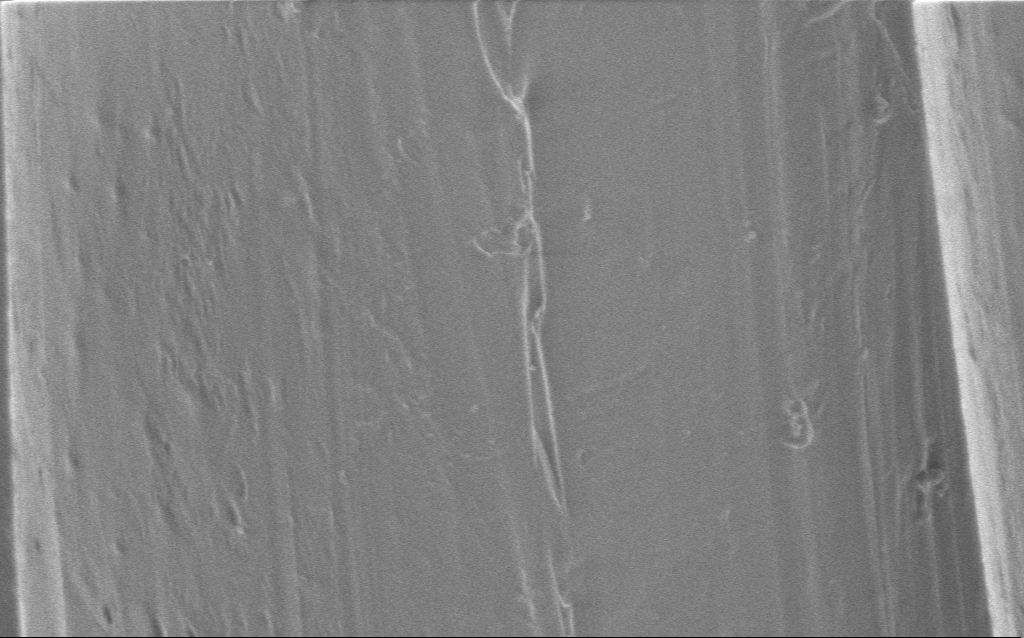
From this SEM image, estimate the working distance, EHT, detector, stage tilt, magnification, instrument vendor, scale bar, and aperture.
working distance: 4 mm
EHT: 1 kV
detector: InLens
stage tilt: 0°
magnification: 17.04 K X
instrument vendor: Zeiss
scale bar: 2000 nm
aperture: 30 µm